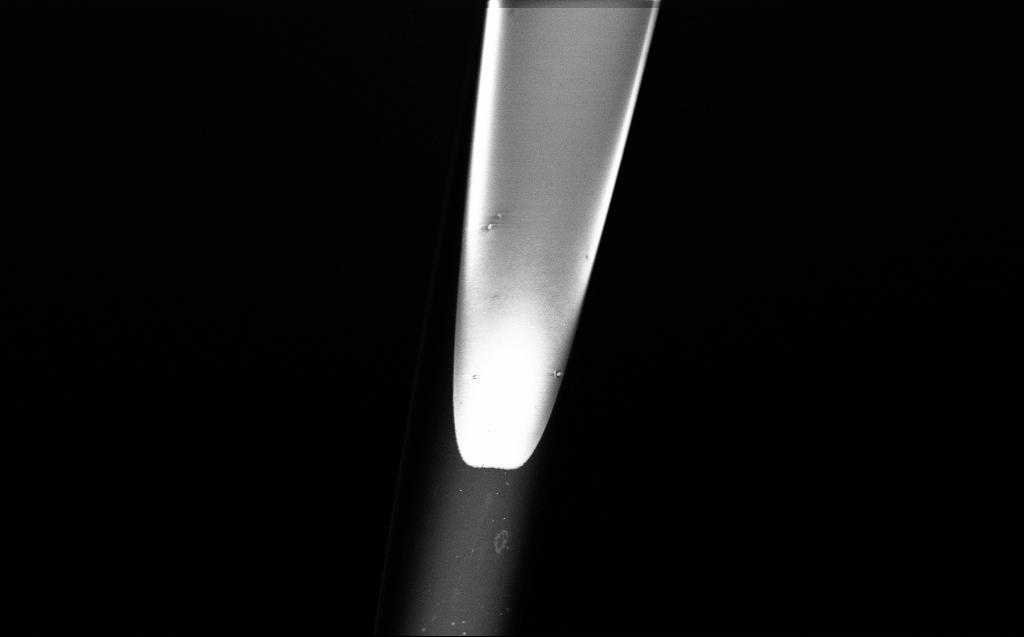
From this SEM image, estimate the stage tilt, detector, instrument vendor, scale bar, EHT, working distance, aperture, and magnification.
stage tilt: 45°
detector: InLens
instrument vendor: Zeiss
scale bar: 10000 nm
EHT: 2 kV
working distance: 4 mm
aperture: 30 µm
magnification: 5 K X